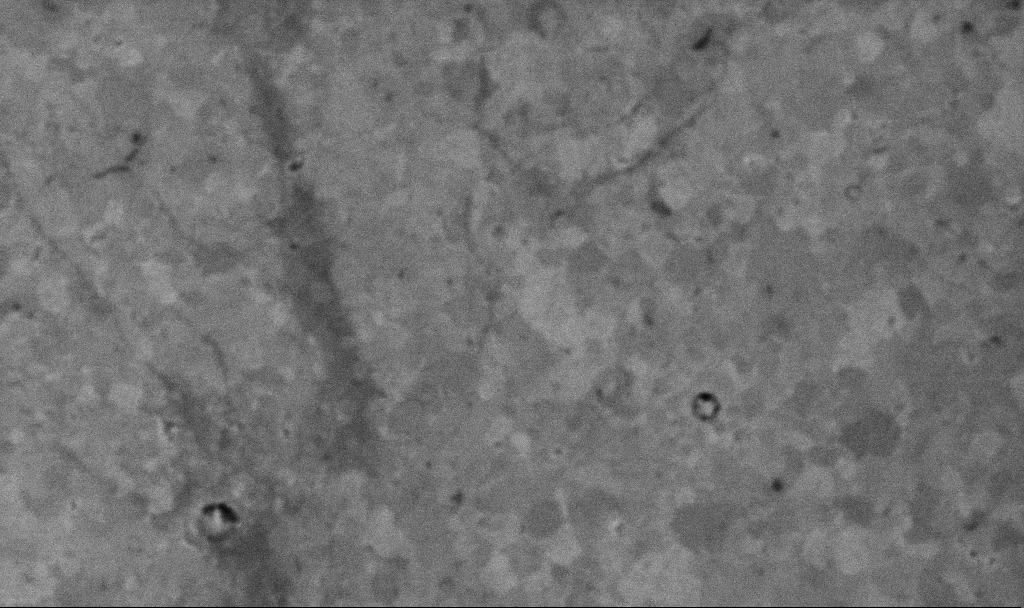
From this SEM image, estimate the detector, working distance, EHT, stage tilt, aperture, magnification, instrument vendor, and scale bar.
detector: InLens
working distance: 3.1 mm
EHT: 10 kV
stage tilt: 0°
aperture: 30 µm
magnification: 50.56 K X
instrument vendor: Zeiss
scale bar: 1000 nm